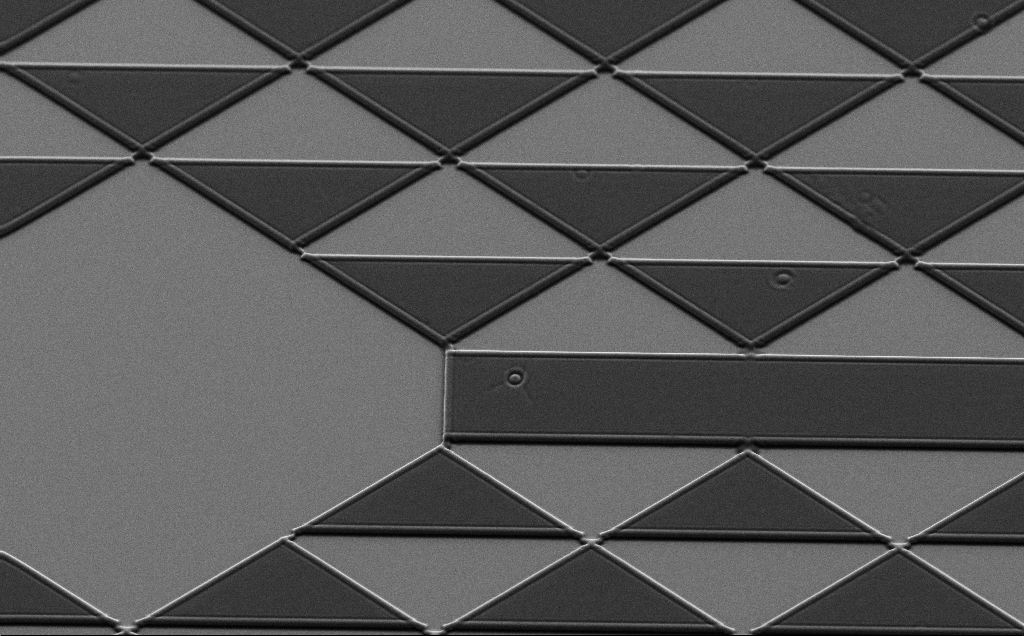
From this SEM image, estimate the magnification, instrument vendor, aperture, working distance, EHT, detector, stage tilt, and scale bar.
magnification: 1.21 K X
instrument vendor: Zeiss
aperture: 30 µm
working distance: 6 mm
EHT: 7 kV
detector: SE2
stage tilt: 35°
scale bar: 10000 nm